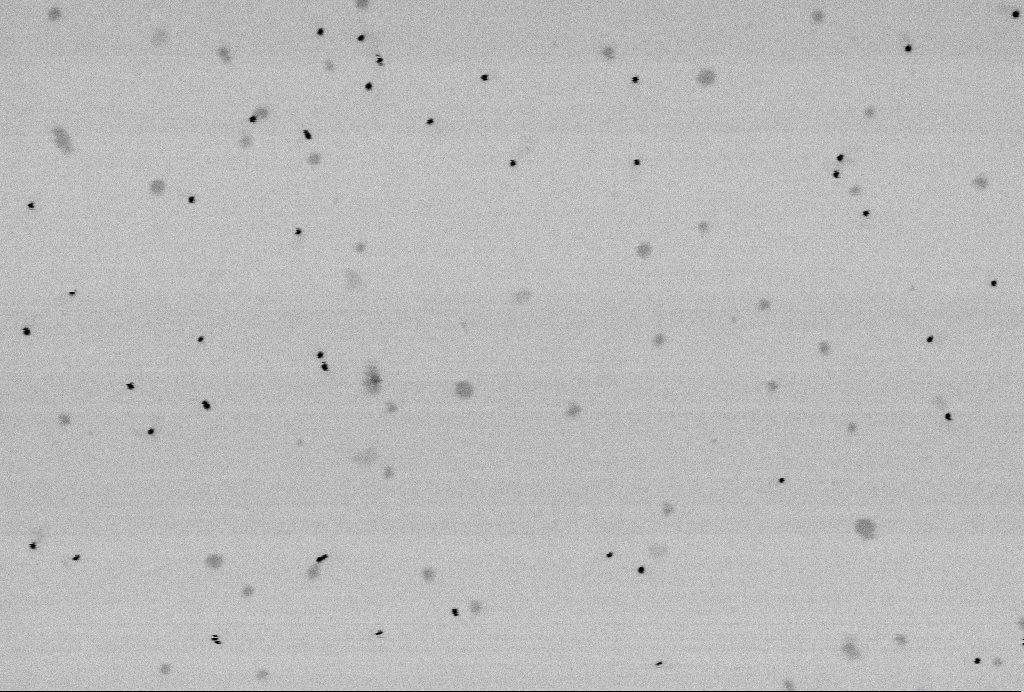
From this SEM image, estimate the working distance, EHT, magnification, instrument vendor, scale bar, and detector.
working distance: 4.9 mm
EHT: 2 kV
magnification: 85.74 K X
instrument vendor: Zeiss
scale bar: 200 nm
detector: SE2